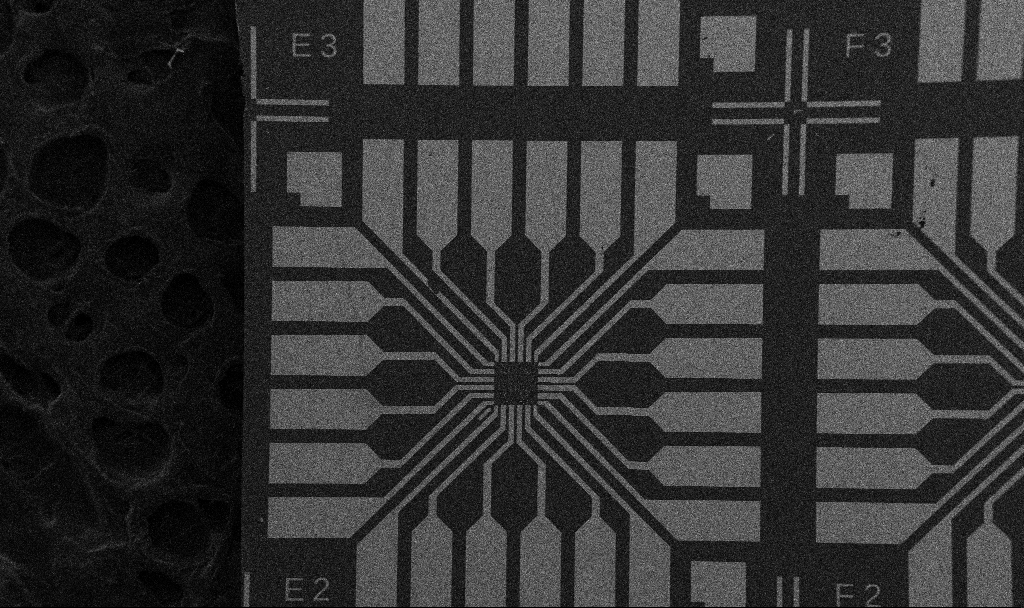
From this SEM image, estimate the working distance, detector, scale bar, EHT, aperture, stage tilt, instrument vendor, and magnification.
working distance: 10.7 mm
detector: SE2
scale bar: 200000 nm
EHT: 5 kV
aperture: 30 µm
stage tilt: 0°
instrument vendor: Zeiss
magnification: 0.1 K X